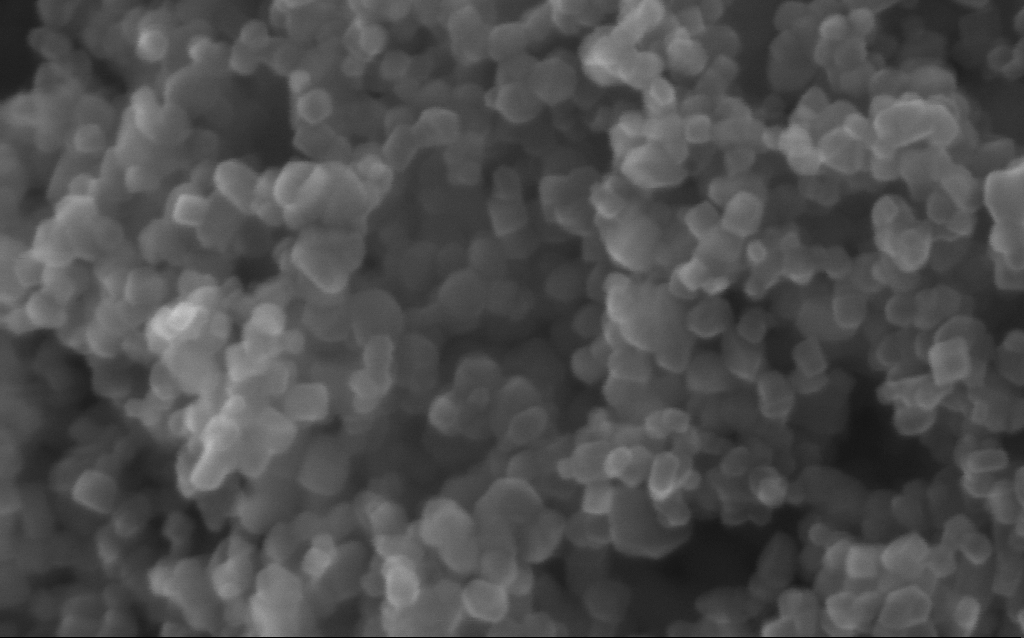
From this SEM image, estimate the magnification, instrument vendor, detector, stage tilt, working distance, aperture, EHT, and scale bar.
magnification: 600 K X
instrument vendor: Zeiss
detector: InLens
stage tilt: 0°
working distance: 2.8 mm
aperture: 30 µm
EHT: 10 kV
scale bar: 100 nm